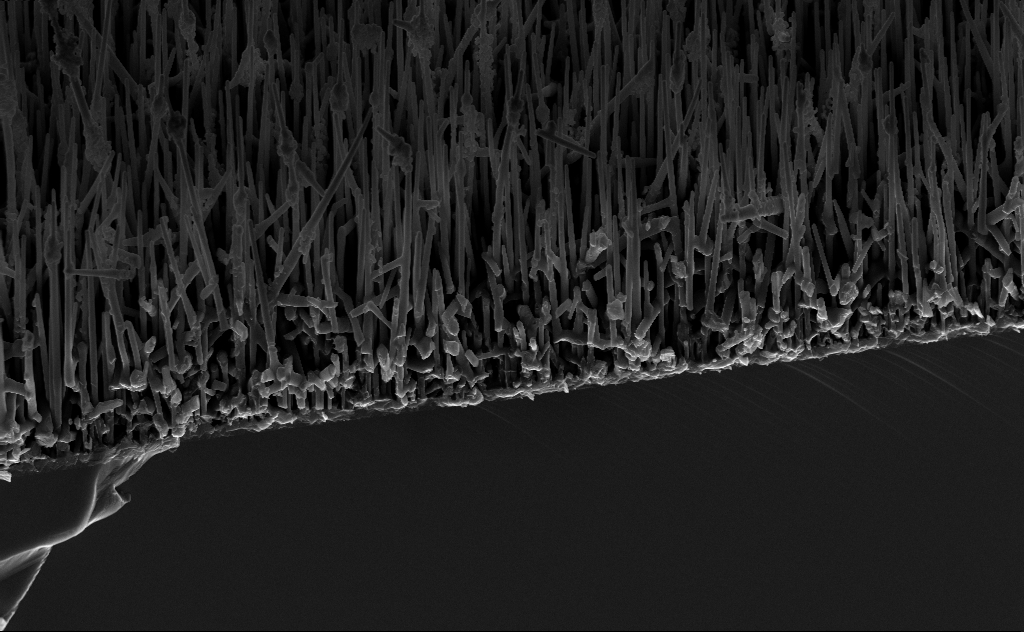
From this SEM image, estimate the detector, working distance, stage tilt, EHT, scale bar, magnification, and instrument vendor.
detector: InLens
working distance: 5 mm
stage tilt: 44.8°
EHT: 10 kV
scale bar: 2000 nm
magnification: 10.32 K X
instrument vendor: Zeiss